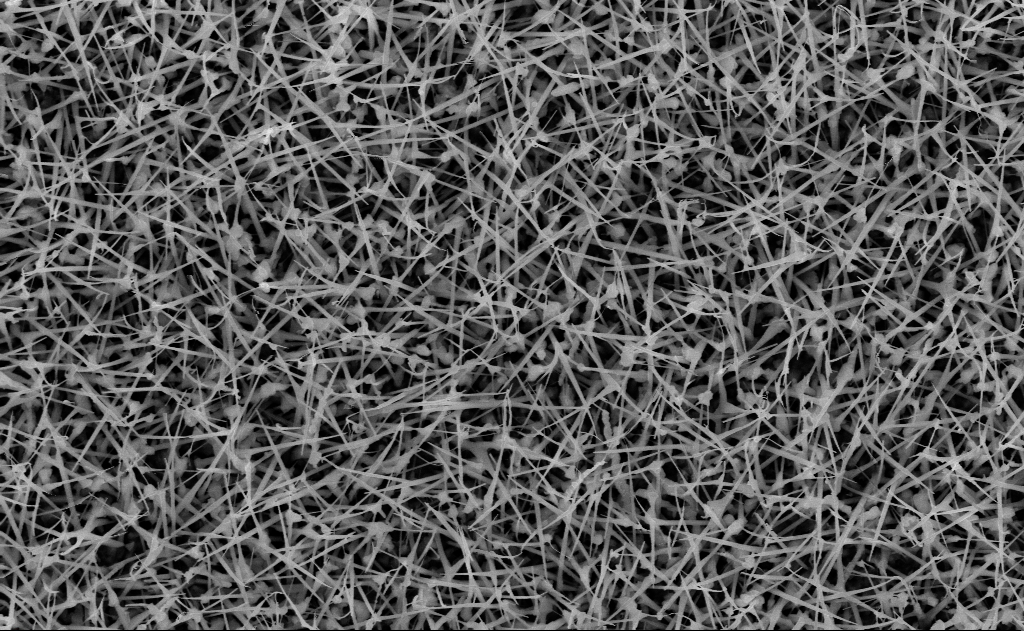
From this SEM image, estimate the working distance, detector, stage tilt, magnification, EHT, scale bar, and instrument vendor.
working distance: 15 mm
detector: InLens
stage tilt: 0°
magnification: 20 K X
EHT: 10 kV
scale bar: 2000 nm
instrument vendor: Zeiss